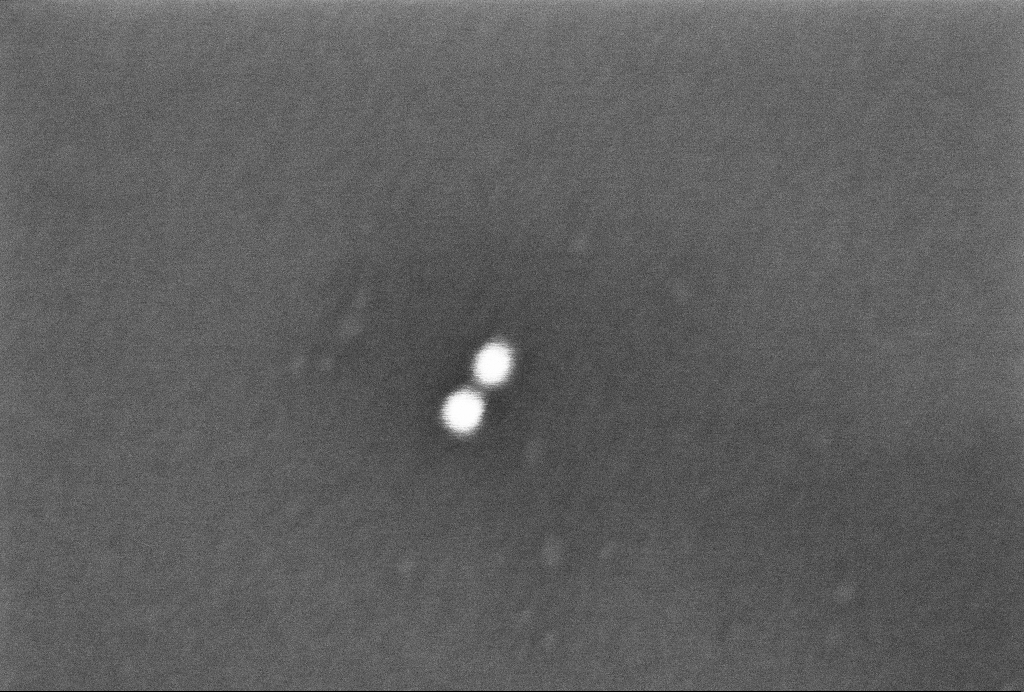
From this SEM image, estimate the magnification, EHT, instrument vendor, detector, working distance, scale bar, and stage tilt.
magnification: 480.82 K X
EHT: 2 kV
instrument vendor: Zeiss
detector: InLens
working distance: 3.3 mm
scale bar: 100 nm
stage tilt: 0°